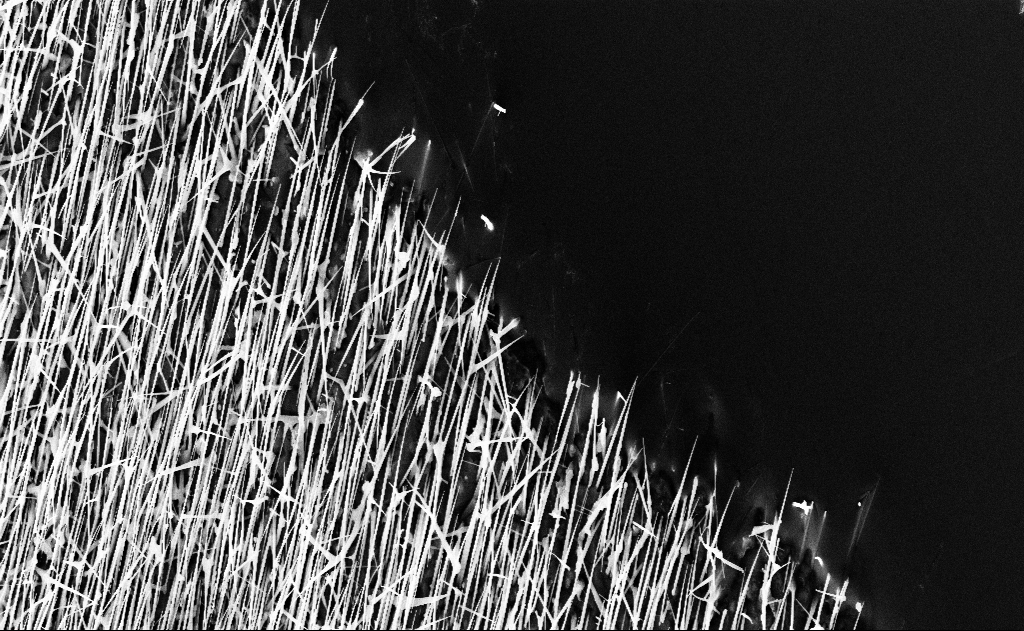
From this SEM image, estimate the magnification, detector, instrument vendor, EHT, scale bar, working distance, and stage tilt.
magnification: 10 K X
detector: InLens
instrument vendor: Zeiss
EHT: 10 kV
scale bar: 2000 nm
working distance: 16 mm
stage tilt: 0°